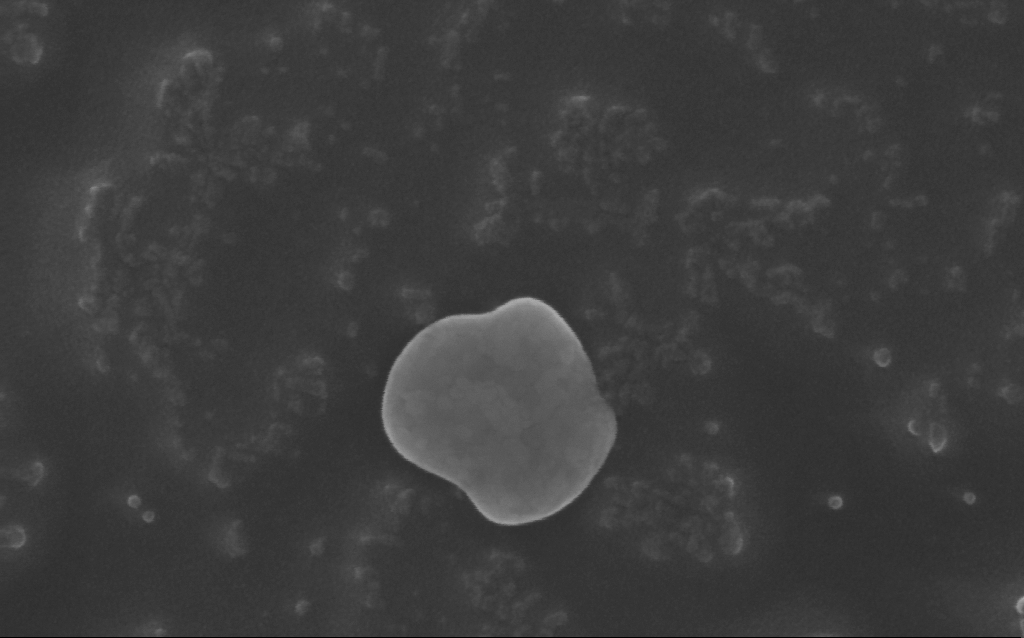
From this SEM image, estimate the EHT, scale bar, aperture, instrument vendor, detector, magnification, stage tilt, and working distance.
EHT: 10 kV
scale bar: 200 nm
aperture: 30 µm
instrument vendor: Zeiss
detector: InLens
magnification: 307.01 K X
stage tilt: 0°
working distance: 5 mm